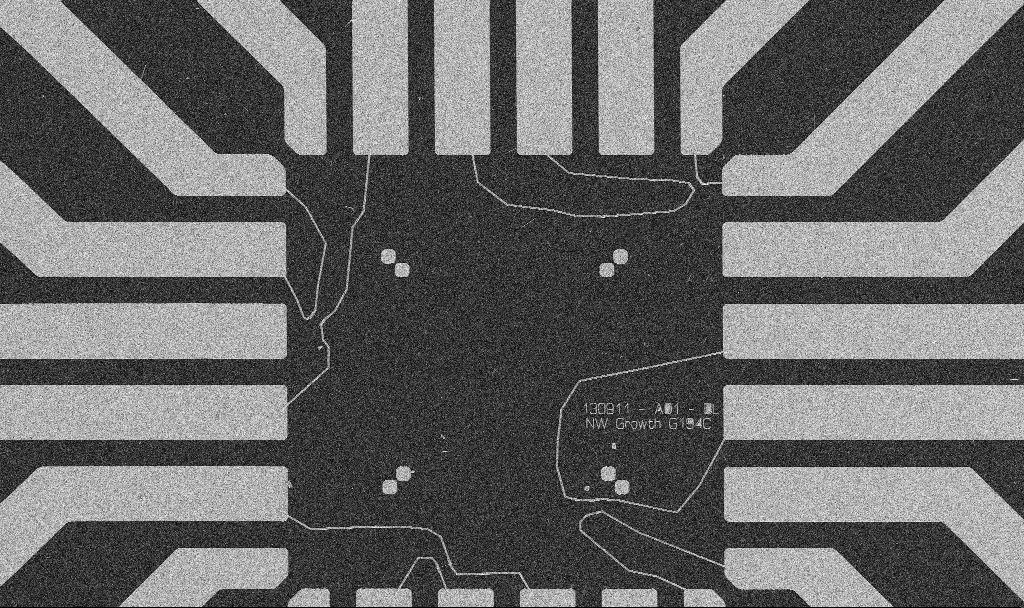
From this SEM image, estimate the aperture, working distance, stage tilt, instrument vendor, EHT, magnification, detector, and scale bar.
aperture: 30 µm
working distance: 10.6 mm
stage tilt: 0°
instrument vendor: Zeiss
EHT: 5 kV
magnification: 1 K X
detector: SE2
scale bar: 20000 nm